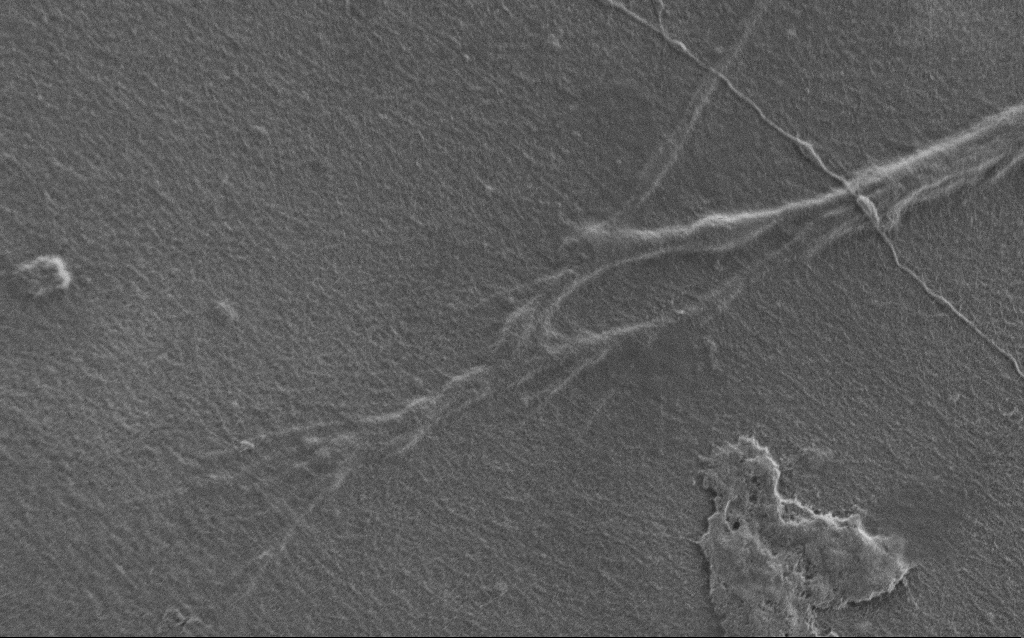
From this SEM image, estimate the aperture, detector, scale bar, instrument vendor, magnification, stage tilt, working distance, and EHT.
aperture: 30 µm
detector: SE2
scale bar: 2000 nm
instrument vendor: Zeiss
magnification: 7.5 K X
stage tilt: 0°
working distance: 6 mm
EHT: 0.9 kV